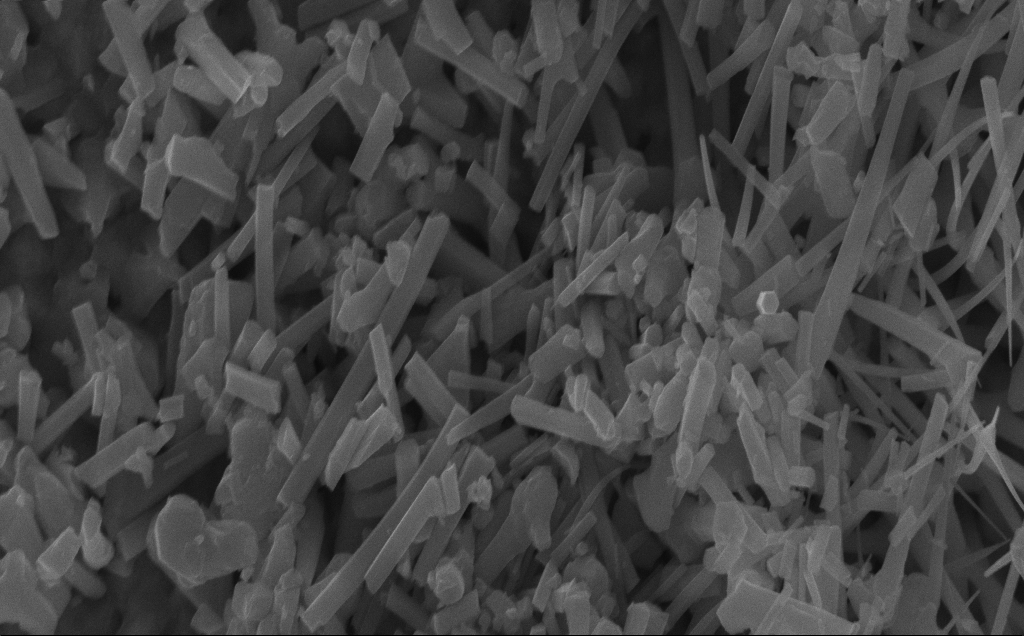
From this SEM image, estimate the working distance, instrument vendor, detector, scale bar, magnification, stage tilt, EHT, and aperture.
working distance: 7 mm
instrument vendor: Zeiss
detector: InLens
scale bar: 1000 nm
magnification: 51.06 K X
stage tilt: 0°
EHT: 10 kV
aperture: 30 µm